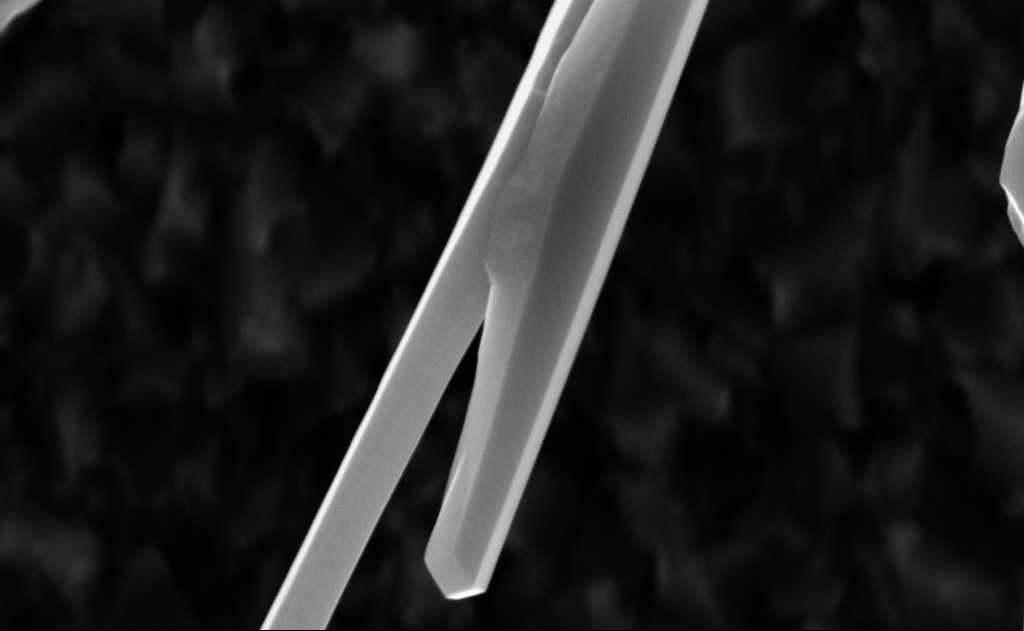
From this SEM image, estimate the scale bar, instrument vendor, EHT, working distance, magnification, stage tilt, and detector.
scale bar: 200 nm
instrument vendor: Zeiss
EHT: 10 kV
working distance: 6 mm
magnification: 80 K X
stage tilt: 0°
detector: InLens